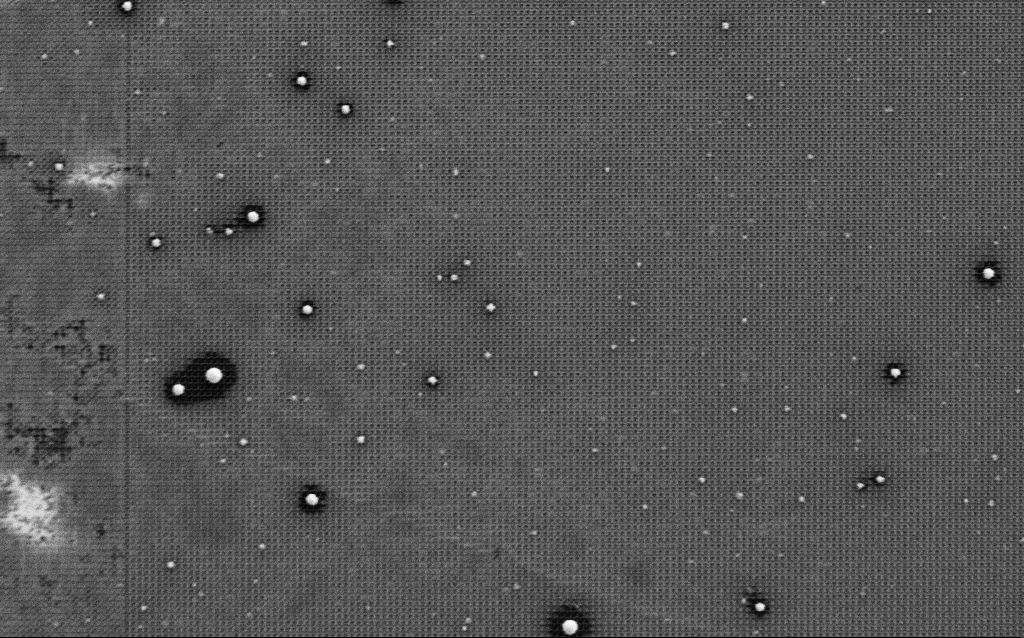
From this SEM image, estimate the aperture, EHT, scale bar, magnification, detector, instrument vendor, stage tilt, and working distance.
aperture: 30 µm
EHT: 1.5 kV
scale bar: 10000 nm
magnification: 5.08 K X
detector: SE2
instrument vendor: Zeiss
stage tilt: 0°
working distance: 8 mm